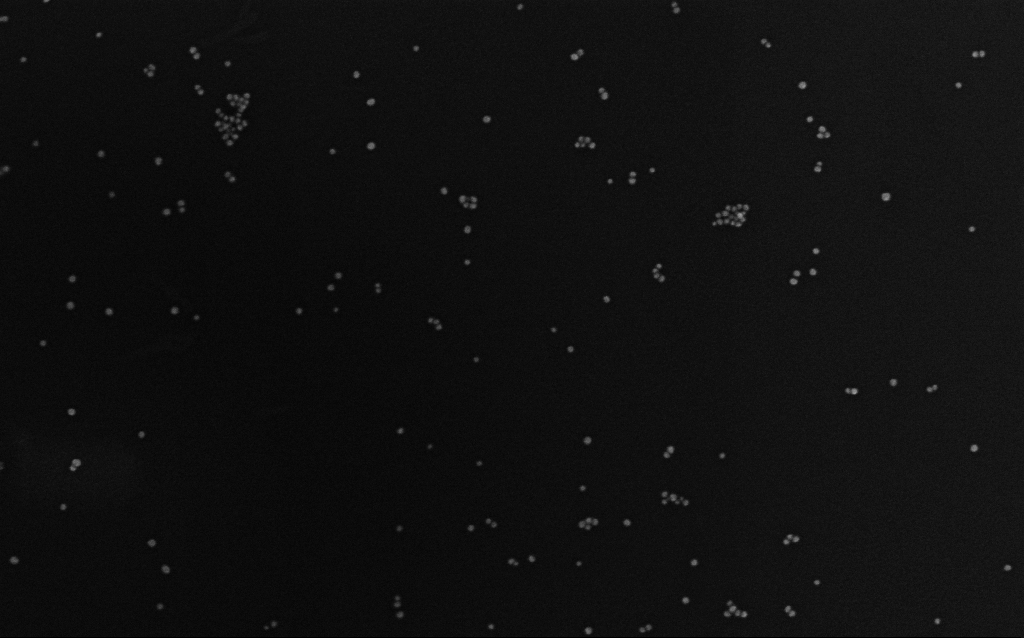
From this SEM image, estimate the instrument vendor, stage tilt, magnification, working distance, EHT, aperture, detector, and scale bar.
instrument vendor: Zeiss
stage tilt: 0°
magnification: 169.61 K X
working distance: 2.7 mm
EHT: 8 kV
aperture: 30 µm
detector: InLens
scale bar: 100 nm